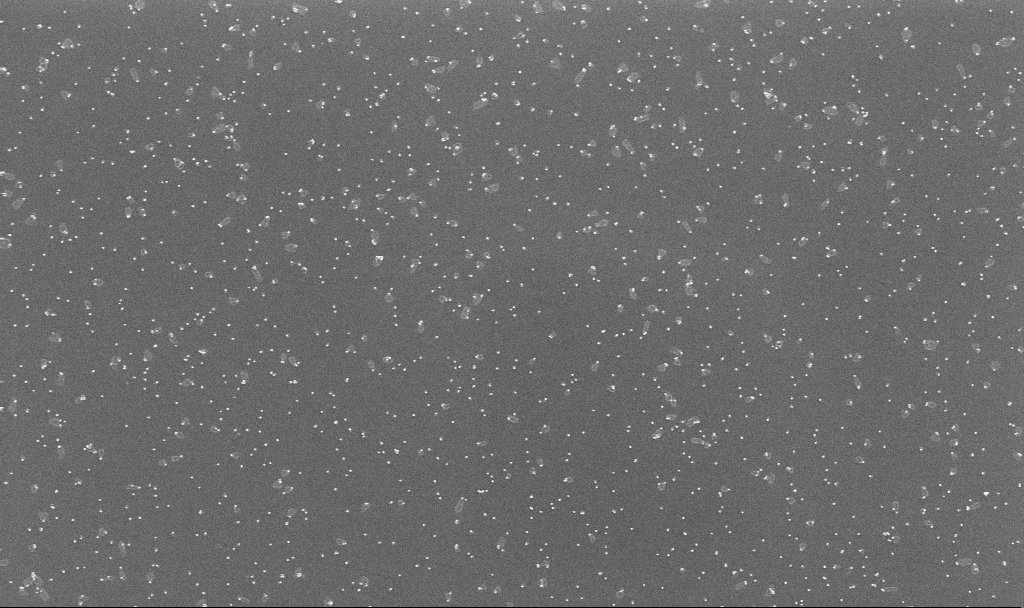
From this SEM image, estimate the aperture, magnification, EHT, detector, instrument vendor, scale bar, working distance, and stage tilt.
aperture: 30 µm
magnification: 70 K X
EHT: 10 kV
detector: InLens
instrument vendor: Zeiss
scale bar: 1000 nm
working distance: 3.3 mm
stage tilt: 0°